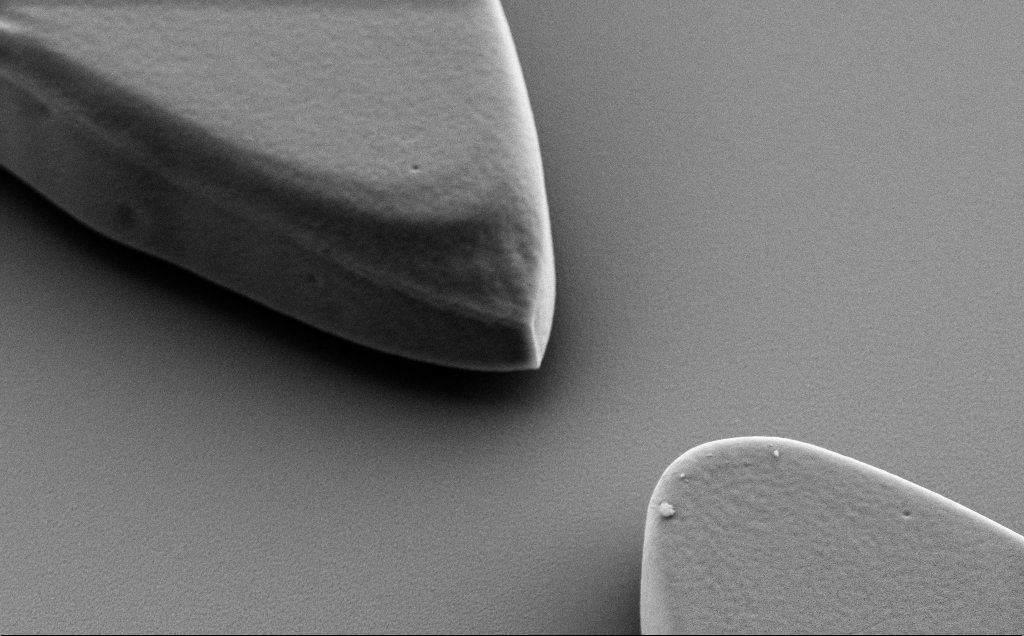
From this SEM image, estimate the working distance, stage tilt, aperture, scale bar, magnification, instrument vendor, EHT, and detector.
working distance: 9 mm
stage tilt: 40°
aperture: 30 µm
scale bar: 2000 nm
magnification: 13.6 K X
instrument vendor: Zeiss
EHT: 5 kV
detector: SE2